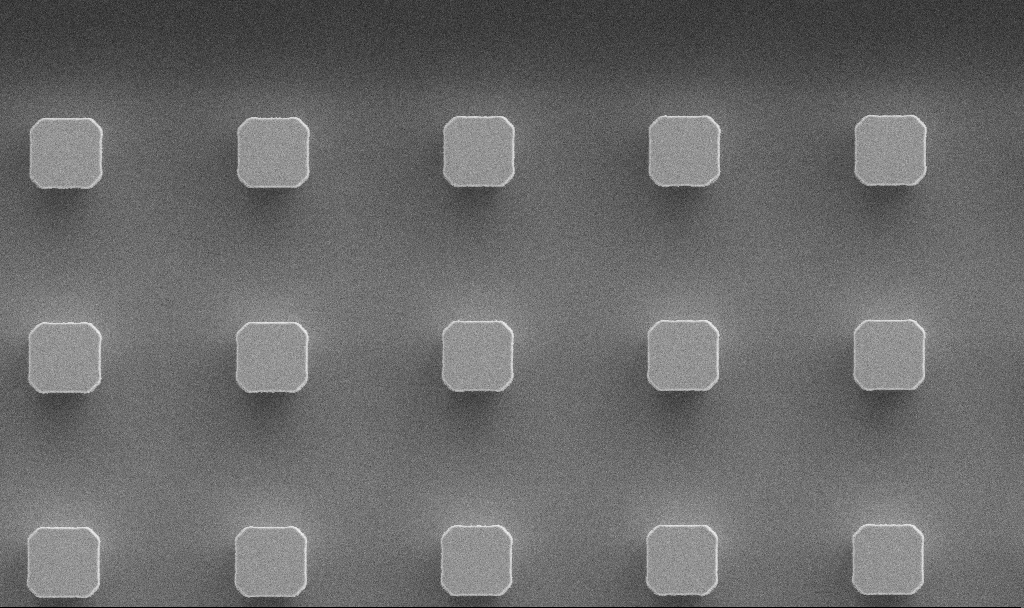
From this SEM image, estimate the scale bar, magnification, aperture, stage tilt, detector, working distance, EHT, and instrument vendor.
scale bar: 10000 nm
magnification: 2.52 K X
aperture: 30 µm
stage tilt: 0°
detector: SE2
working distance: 8.8 mm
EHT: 5 kV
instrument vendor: Zeiss